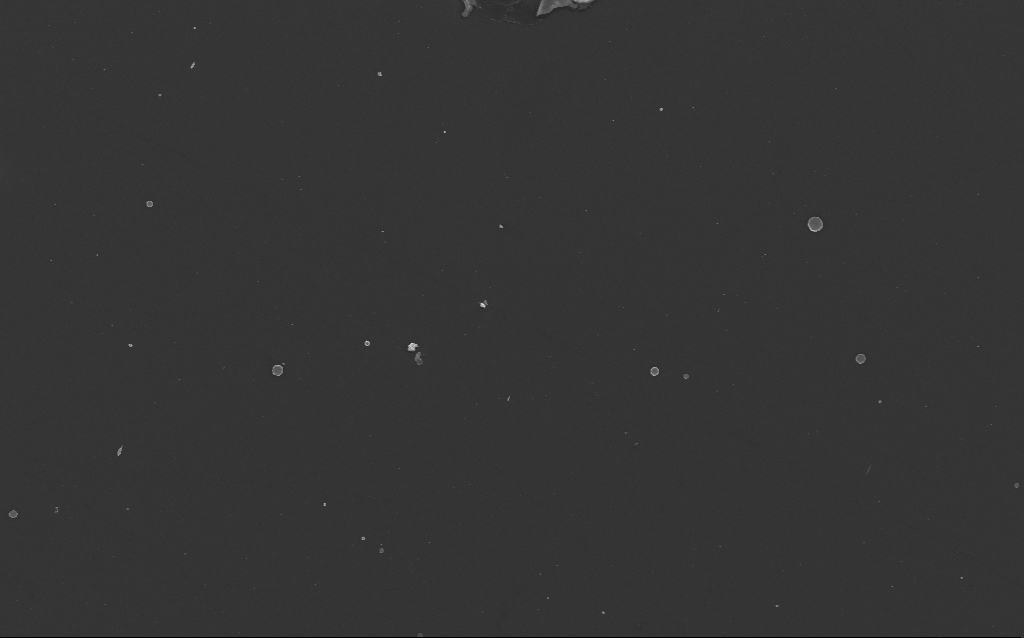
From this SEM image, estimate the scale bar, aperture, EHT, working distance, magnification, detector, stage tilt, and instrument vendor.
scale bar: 10000 nm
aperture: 30 µm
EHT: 10 kV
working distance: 3 mm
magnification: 3.72 K X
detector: InLens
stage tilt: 0°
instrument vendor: Zeiss